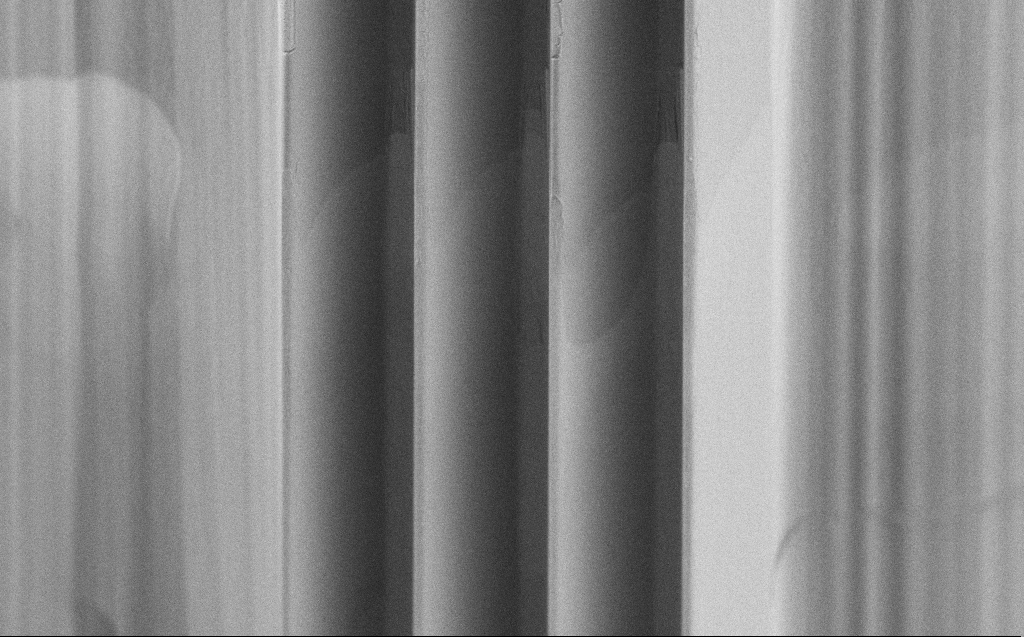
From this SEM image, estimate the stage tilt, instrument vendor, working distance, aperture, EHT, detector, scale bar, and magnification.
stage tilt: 45°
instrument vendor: Zeiss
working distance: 5 mm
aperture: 30 µm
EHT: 5 kV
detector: SE2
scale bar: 10000 nm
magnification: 4.34 K X